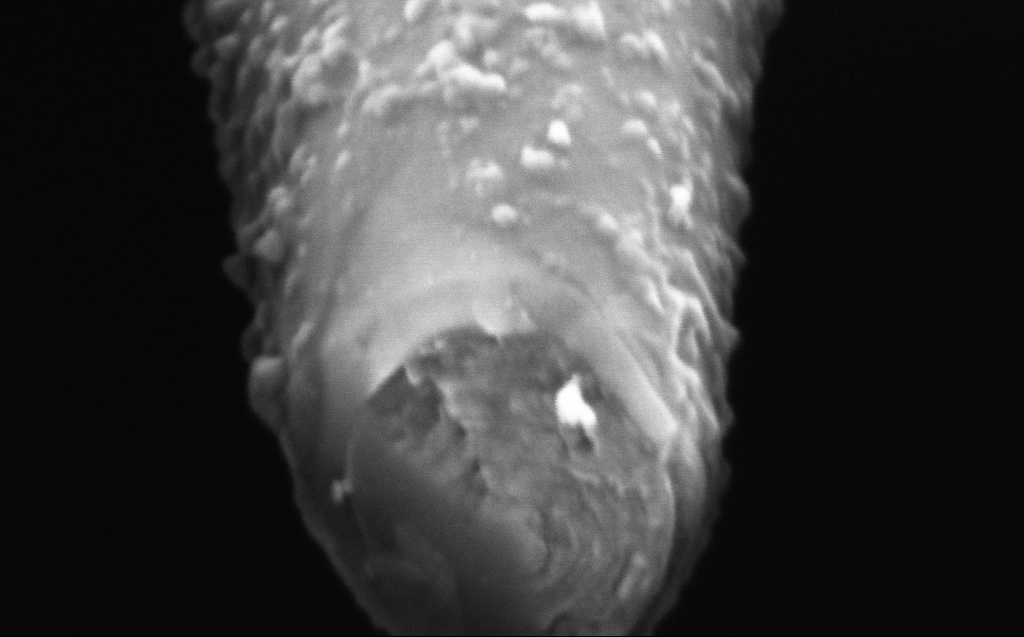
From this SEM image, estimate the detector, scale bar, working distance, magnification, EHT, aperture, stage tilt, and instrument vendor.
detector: InLens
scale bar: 100 nm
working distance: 4 mm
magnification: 307.79 K X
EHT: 2 kV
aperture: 30 µm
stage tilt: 45°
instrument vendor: Zeiss